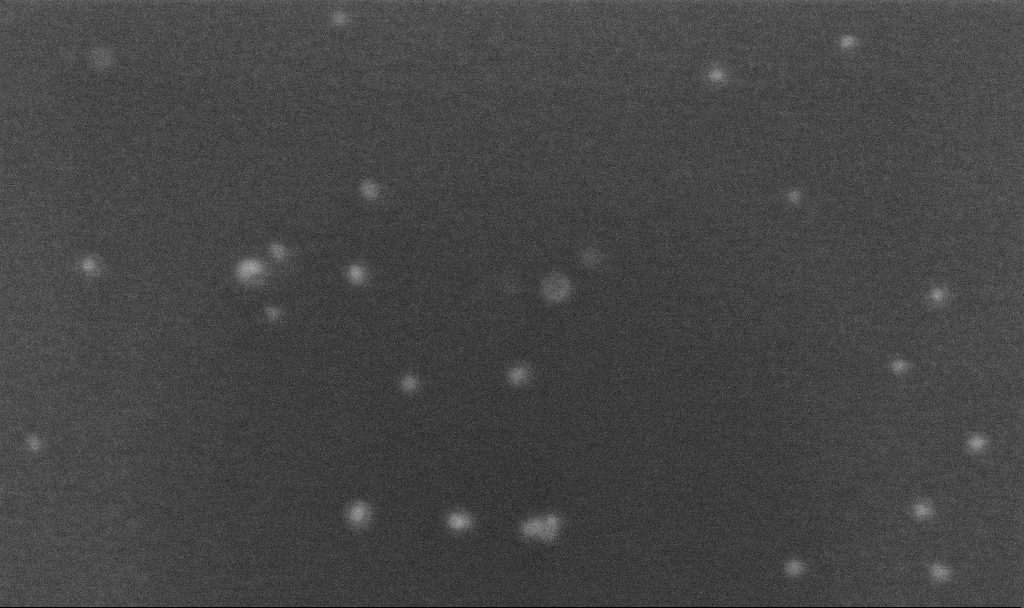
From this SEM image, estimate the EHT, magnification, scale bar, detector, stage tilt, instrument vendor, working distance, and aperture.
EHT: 5 kV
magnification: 500 K X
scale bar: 100 nm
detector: InLens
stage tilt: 0°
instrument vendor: Zeiss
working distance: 3.2 mm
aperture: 30 µm